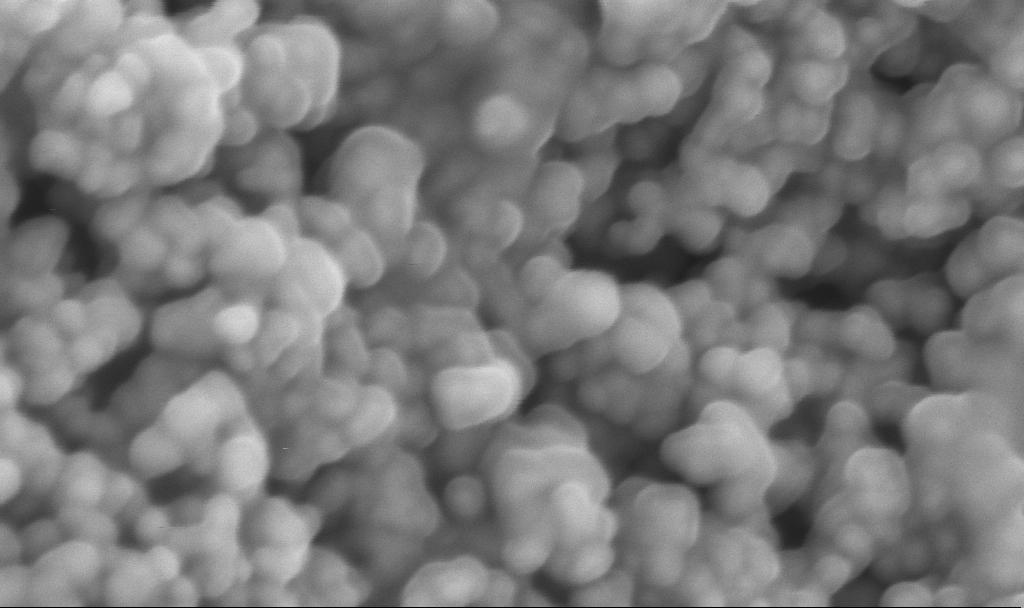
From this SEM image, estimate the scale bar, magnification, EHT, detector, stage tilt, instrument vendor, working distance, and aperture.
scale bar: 200 nm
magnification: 325.68 K X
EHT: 3 kV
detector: InLens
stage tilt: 0°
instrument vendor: Zeiss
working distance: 2.5 mm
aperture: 30 µm